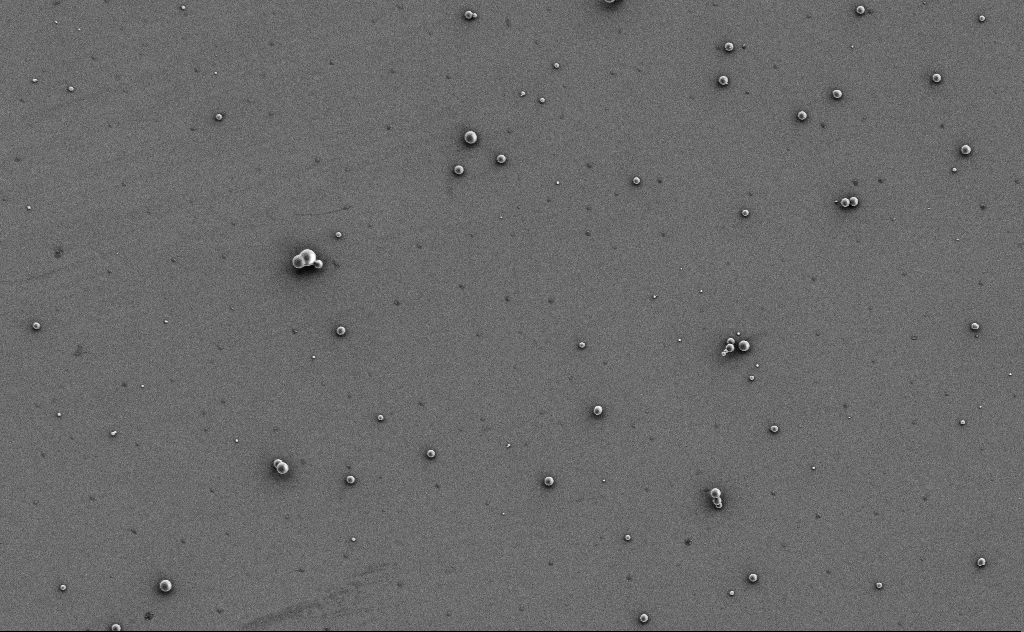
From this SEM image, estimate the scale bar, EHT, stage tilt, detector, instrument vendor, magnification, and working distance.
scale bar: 10000 nm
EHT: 3 kV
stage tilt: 0°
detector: SE2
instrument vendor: Zeiss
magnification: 3.38 K X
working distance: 11 mm